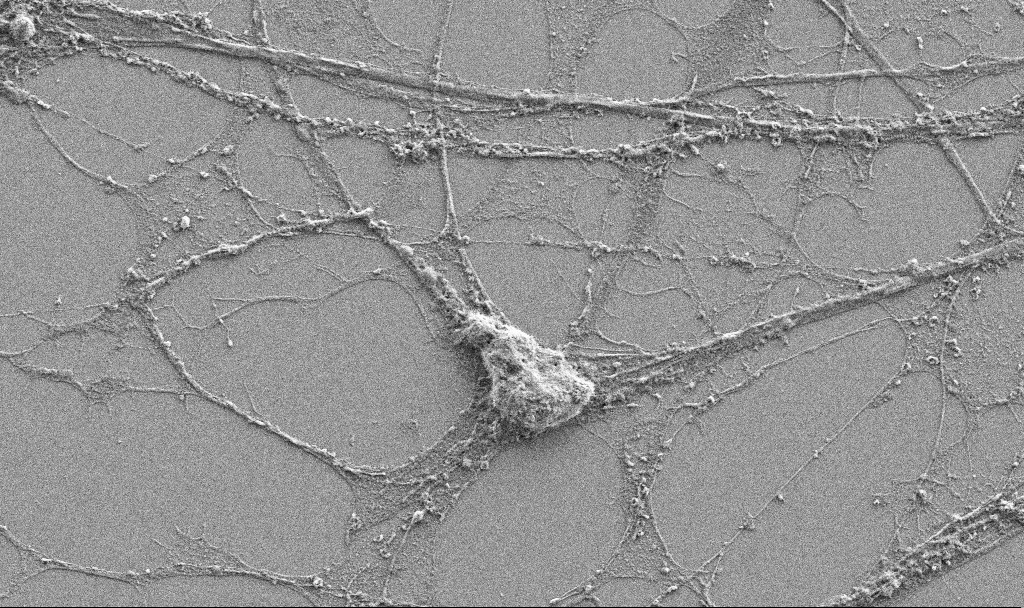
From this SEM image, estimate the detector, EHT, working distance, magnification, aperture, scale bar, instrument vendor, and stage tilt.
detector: SE2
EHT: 3 kV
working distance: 3.5 mm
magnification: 5 K X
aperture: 30 µm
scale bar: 10000 nm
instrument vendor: Zeiss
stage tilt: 0°